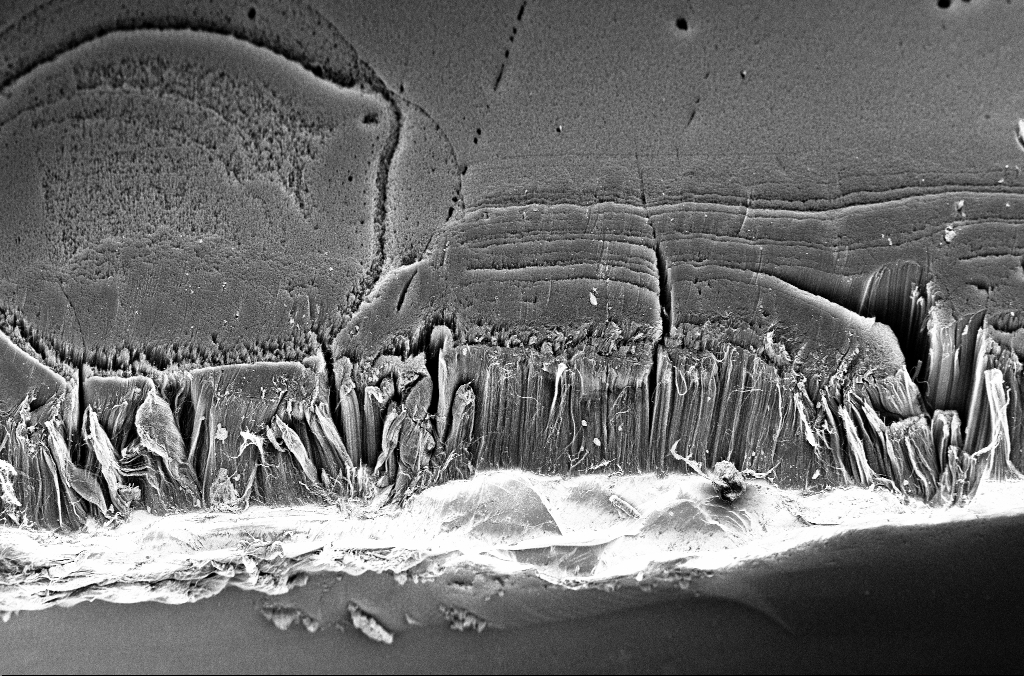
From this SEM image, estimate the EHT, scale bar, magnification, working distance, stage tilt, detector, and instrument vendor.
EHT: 3 kV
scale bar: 100000 nm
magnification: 0.4 K X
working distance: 3.3 mm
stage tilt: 45°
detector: InLens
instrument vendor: Zeiss